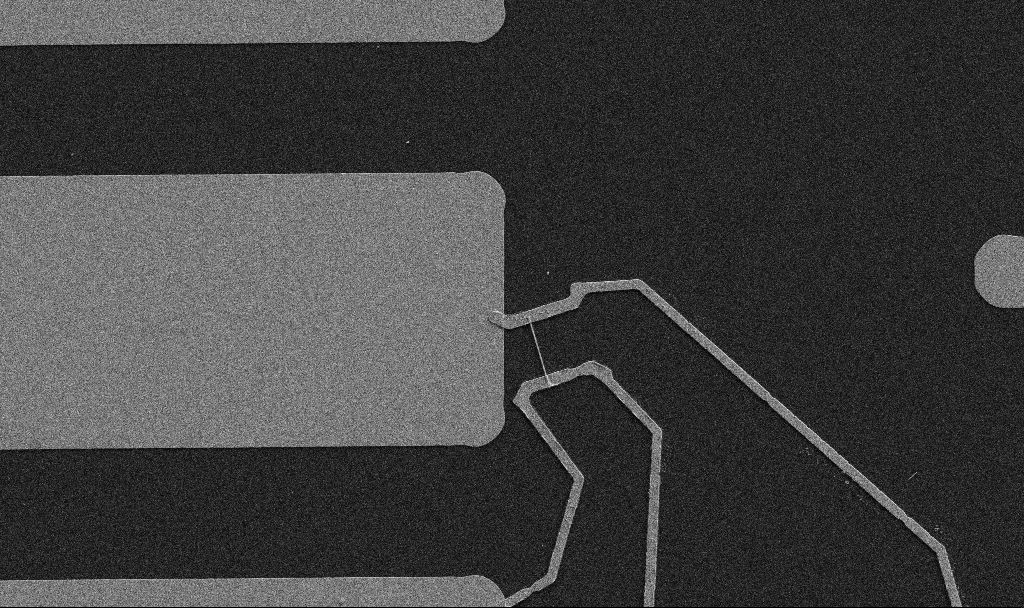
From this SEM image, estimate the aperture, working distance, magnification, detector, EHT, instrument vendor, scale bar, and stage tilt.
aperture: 30 µm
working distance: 10.7 mm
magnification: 5 K X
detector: SE2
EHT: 5 kV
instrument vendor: Zeiss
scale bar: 10000 nm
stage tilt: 0°